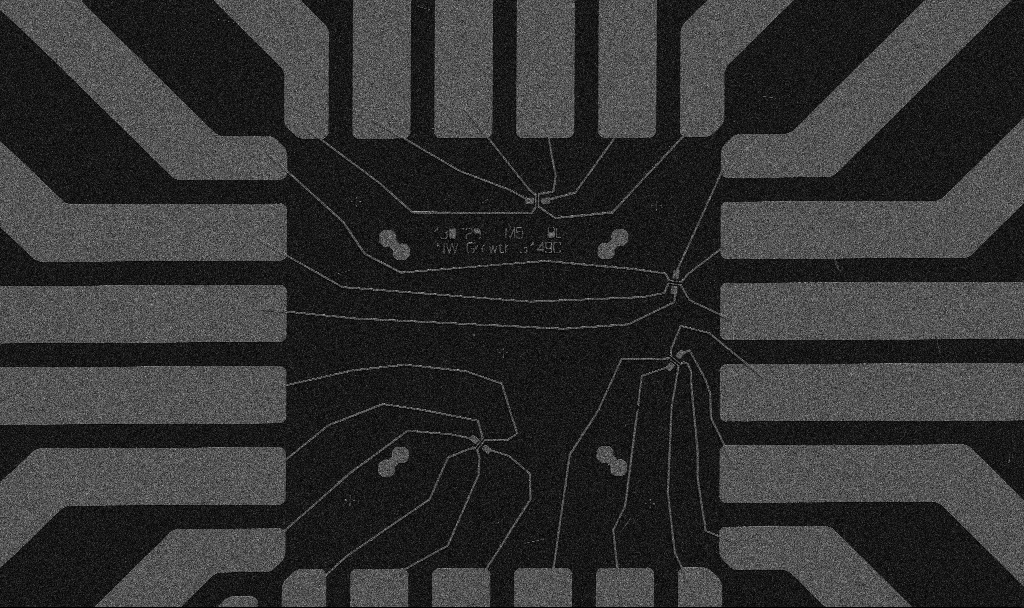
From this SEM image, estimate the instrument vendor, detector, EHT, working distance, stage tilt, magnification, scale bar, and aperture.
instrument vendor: Zeiss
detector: SE2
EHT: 5 kV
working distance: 10.7 mm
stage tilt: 0°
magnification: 1 K X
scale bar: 20000 nm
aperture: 30 µm